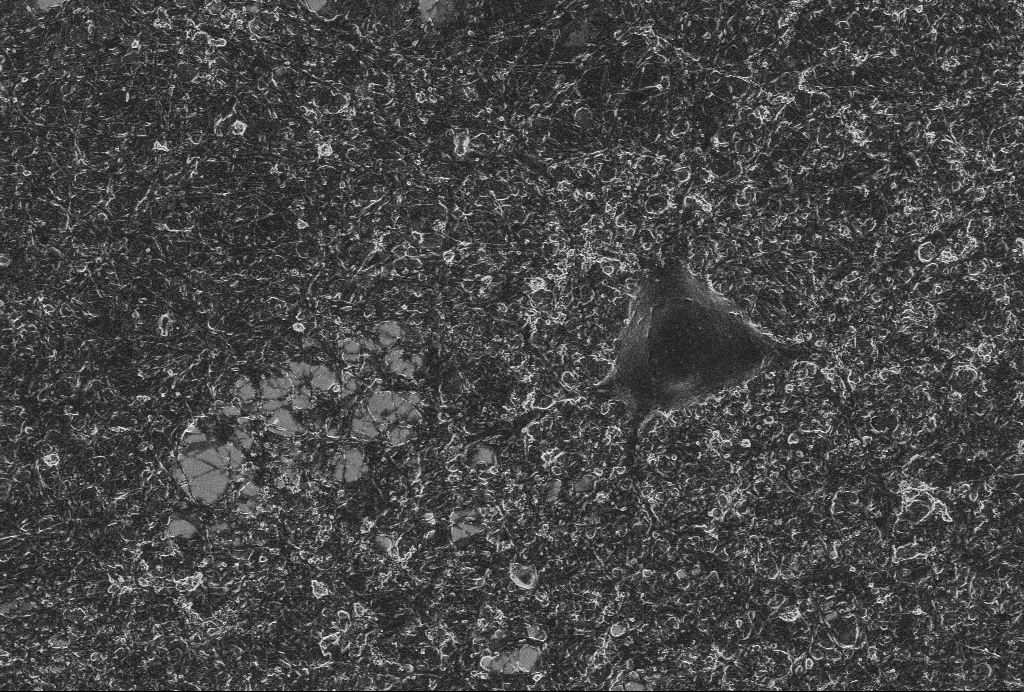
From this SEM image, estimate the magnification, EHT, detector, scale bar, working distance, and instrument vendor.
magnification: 4 K X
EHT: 2 kV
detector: InLens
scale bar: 10000 nm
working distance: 6 mm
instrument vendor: Zeiss